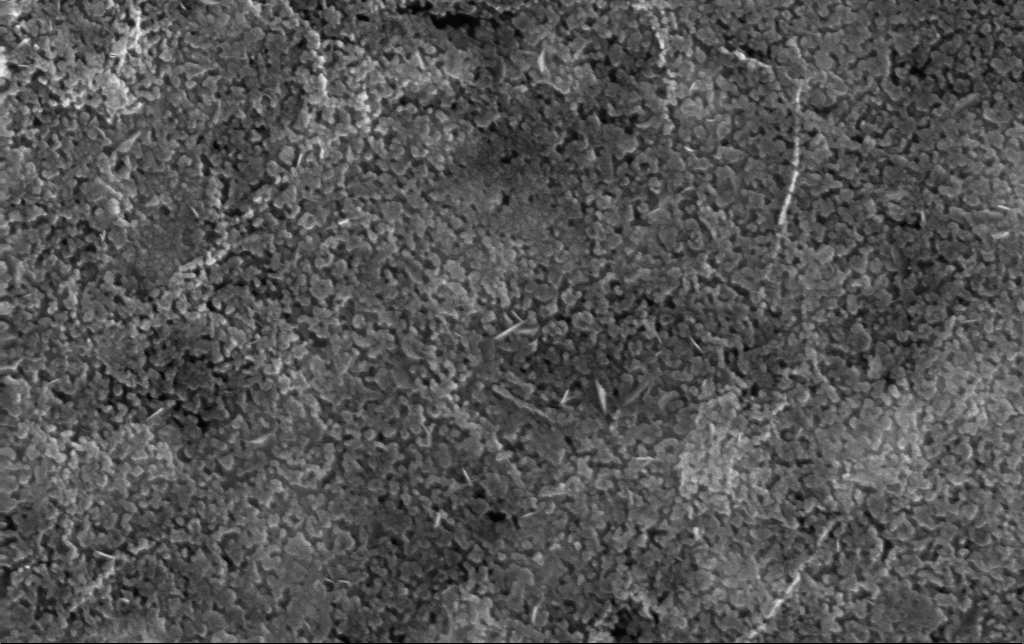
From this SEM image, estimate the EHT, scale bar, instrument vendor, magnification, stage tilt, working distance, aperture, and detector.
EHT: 10 kV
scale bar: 100 nm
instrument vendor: Zeiss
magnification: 150 K X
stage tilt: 0°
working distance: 3 mm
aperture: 30 µm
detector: InLens